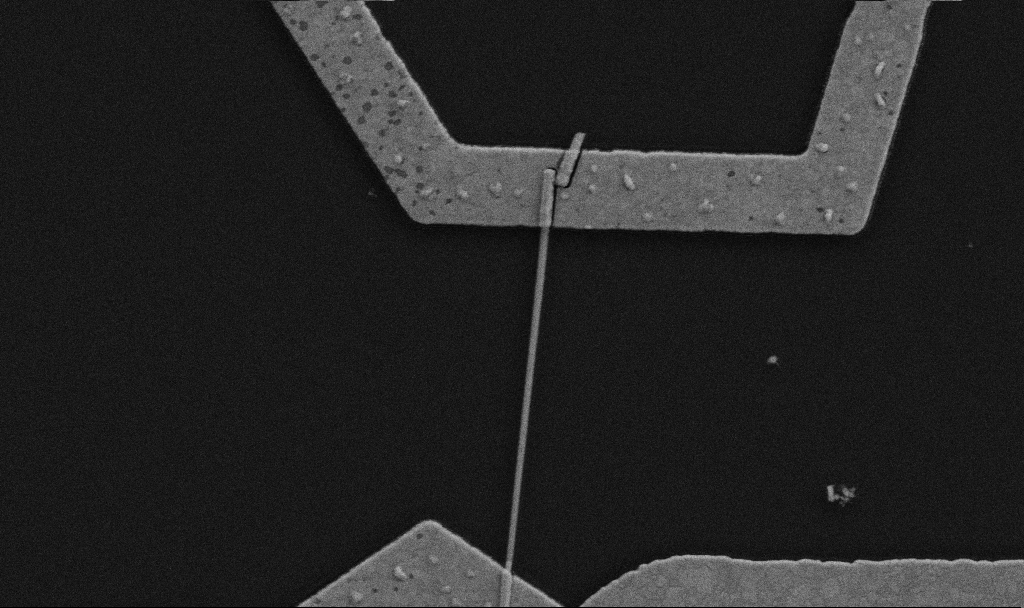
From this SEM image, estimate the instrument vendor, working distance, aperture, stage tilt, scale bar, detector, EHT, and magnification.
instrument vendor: Zeiss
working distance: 10.7 mm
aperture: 30 µm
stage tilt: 0°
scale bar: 1000 nm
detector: SE2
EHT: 5 kV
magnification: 30 K X